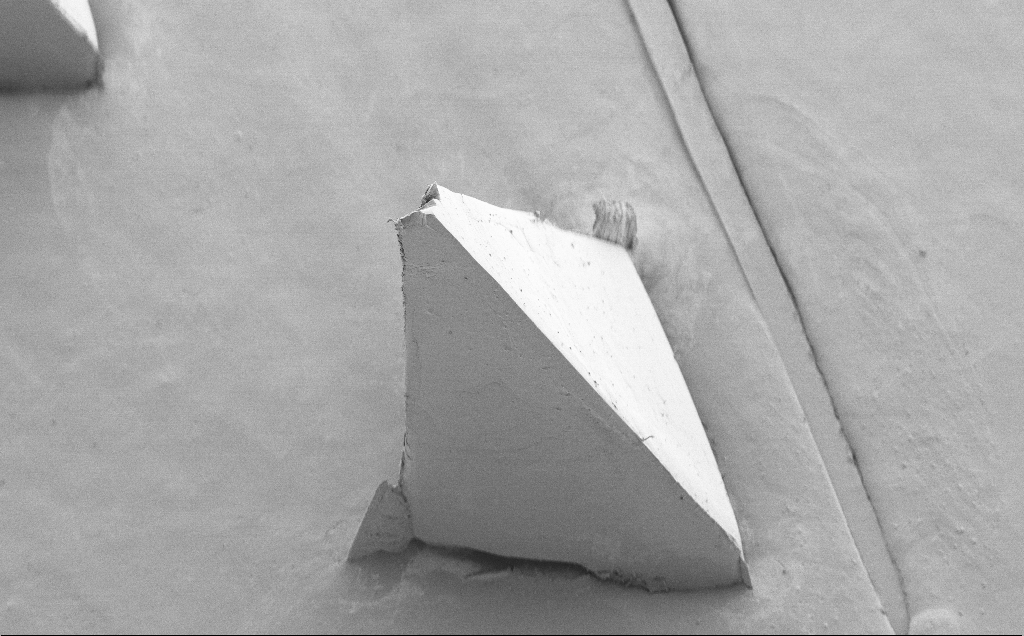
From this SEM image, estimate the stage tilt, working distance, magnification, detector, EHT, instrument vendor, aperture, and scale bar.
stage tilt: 40°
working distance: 8 mm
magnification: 0.199 K X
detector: SE2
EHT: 5 kV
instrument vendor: Zeiss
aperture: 30 µm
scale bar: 200000 nm